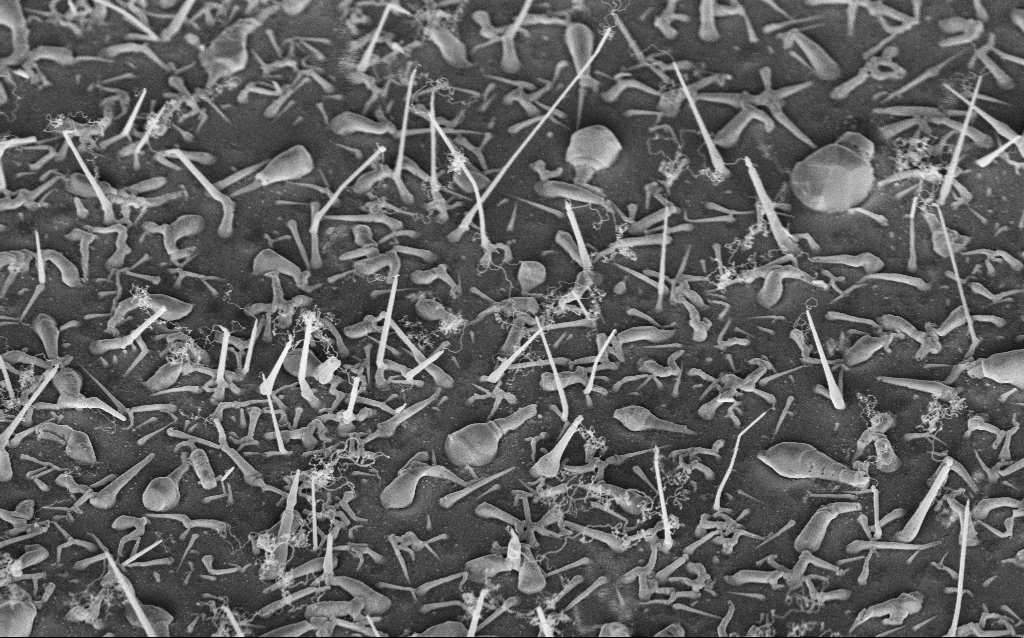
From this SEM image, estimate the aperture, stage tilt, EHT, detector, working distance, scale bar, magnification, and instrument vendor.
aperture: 30 µm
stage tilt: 42°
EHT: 5 kV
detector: InLens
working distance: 8 mm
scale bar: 2000 nm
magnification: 20 K X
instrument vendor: Zeiss